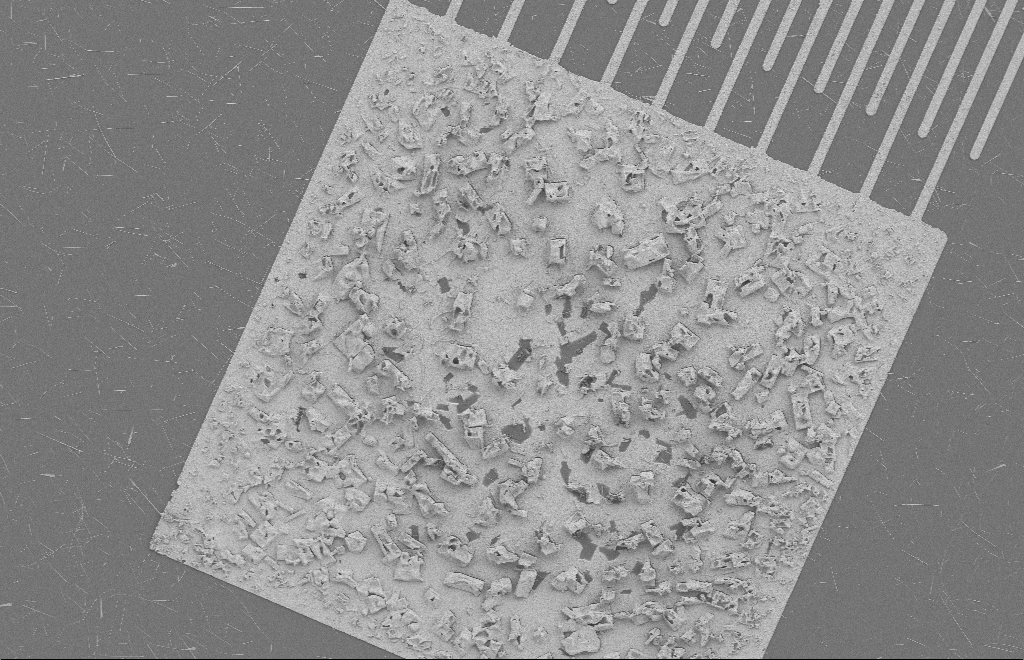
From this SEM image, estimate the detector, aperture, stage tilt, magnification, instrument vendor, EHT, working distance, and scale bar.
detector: SE2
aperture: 20 µm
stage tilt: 0°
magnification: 1.47 K X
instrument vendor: Zeiss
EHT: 2 kV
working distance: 8 mm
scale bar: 10000 nm